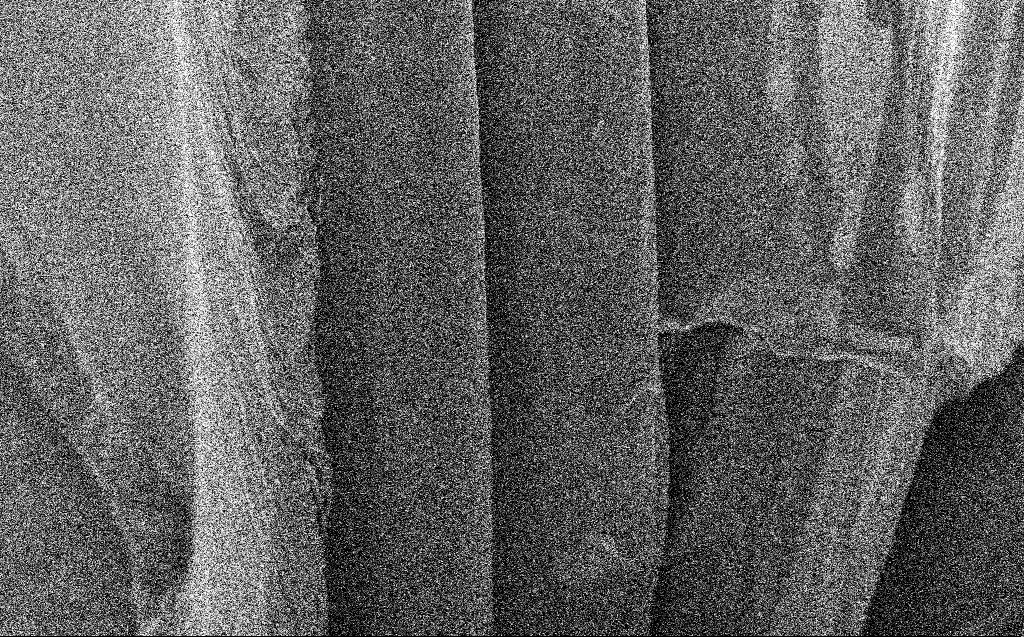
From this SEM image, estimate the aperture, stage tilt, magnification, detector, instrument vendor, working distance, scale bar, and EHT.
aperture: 30 µm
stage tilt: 45°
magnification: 5.97 K X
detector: InLens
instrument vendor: Zeiss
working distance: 9 mm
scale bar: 10000 nm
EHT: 5 kV